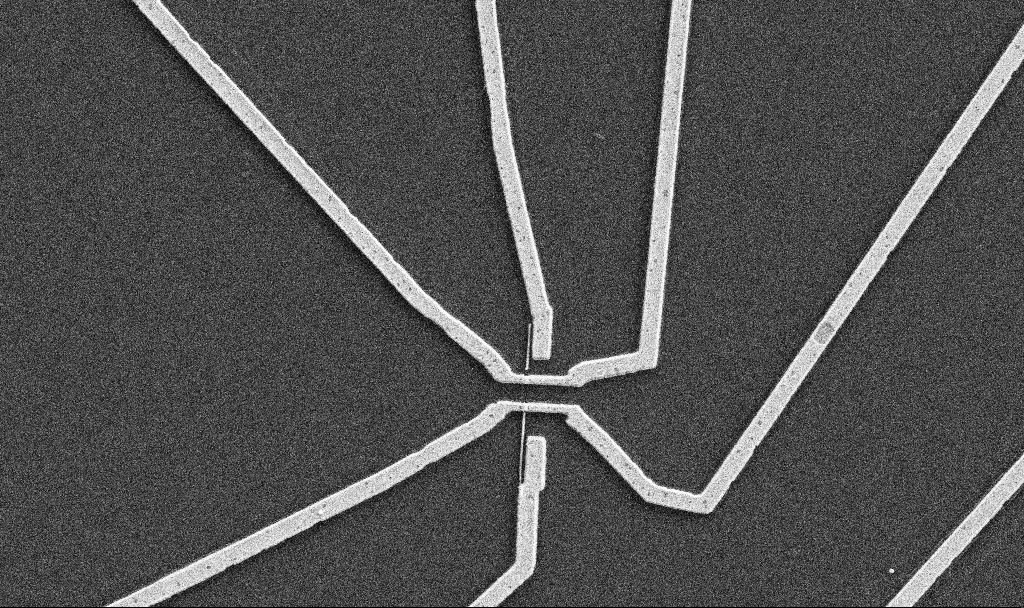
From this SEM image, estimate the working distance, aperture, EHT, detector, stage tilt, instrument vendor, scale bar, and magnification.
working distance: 10.7 mm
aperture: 30 µm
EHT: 5 kV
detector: SE2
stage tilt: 0°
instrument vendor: Zeiss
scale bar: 2000 nm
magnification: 10 K X